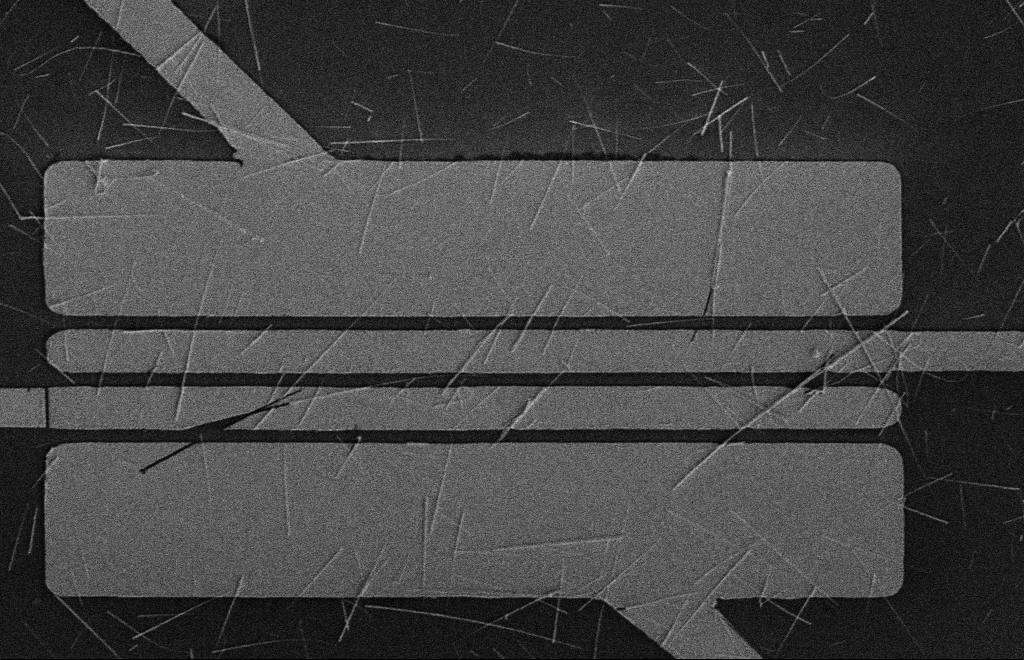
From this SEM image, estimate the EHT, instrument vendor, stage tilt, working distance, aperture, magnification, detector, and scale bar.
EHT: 5 kV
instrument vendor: Zeiss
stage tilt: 0°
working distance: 16 mm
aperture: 10 µm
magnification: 5.17 K X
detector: SE2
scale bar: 2000 nm